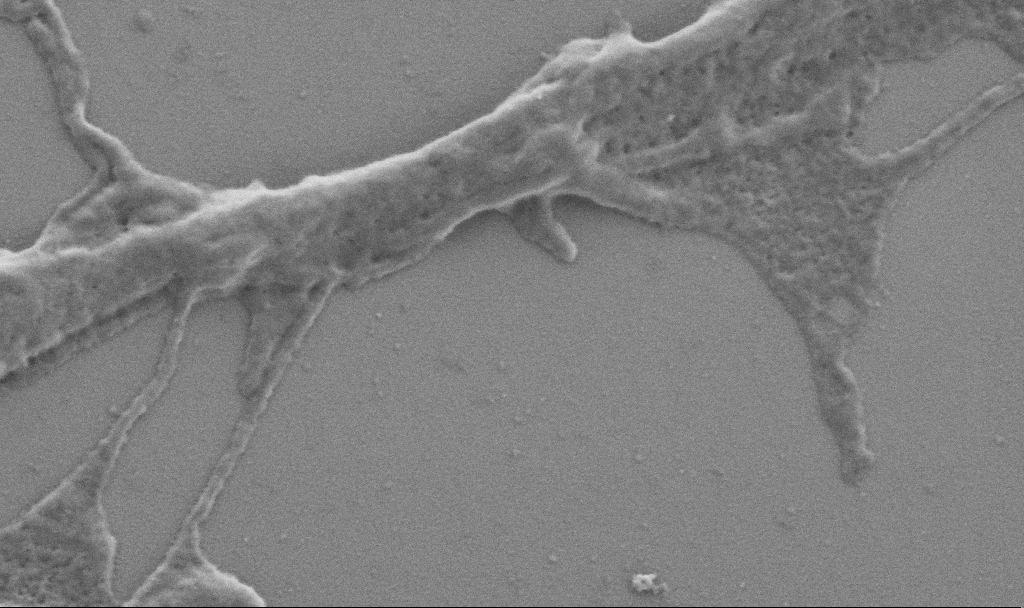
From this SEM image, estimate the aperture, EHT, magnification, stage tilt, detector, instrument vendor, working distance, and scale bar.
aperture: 30 µm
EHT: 0.9 kV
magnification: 50 K X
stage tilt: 0°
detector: SE2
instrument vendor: Zeiss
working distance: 6.9 mm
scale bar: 1000 nm